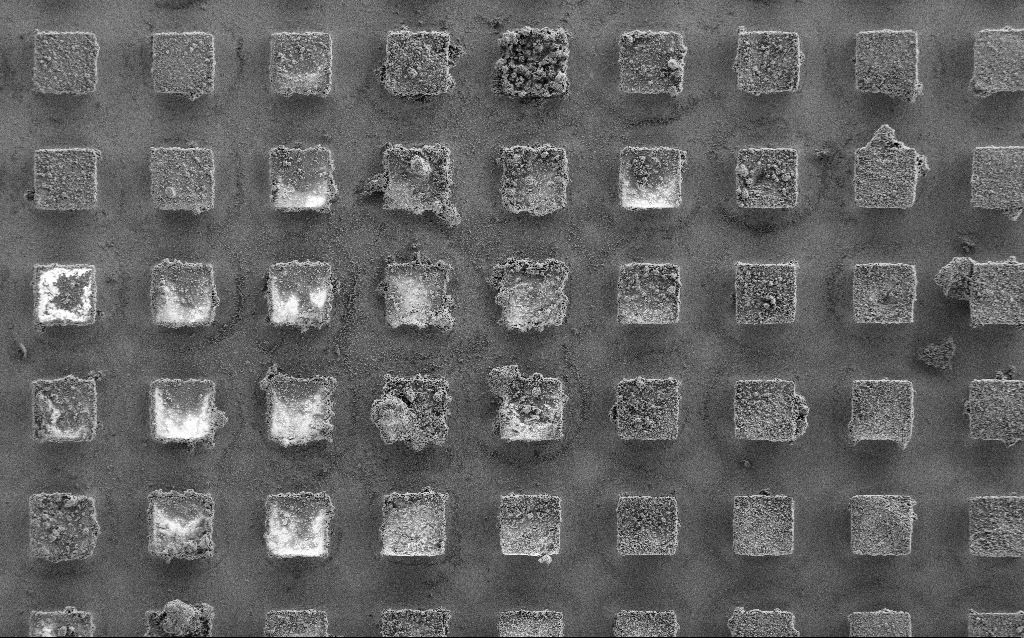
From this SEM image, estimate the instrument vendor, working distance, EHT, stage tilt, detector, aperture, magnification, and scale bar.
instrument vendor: Zeiss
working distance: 5.3 mm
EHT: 2 kV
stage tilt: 0°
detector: SE2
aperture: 30 µm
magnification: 0.432 K X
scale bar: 100000 nm